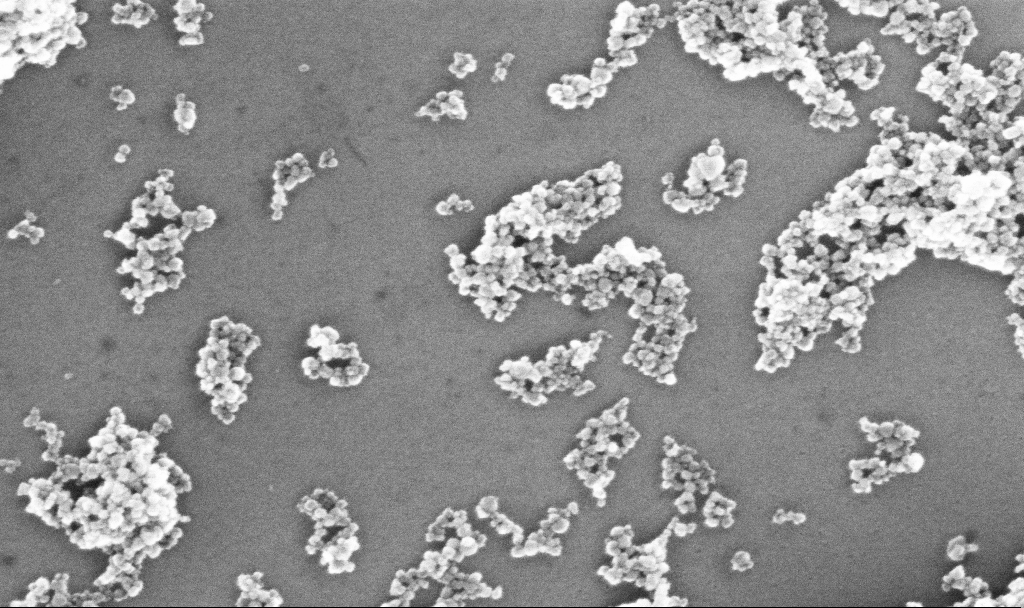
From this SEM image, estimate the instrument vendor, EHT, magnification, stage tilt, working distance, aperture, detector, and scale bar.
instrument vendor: Zeiss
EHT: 10 kV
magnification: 203.45 K X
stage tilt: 0°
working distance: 5.2 mm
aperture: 30 µm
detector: InLens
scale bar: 200 nm